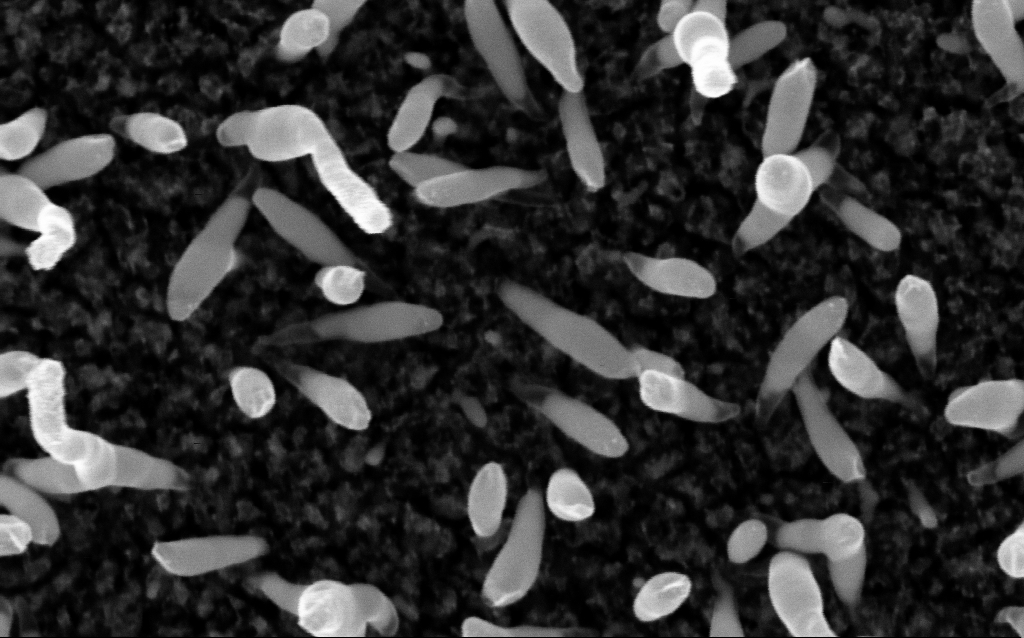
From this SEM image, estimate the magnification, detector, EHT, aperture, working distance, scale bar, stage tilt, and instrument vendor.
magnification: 200 K X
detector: InLens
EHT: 5 kV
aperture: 30 µm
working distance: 2.1 mm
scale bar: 100 nm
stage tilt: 0°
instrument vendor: Zeiss